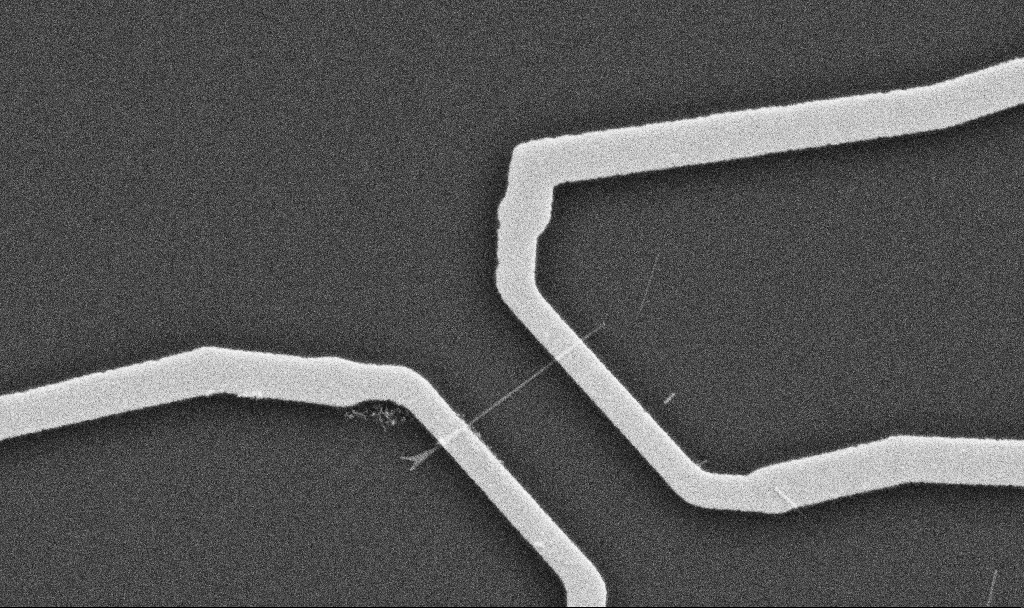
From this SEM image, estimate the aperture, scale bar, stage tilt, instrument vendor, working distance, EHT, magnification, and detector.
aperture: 30 µm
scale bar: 1000 nm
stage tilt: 0°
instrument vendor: Zeiss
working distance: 10.7 mm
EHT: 10 kV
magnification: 20 K X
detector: SE2